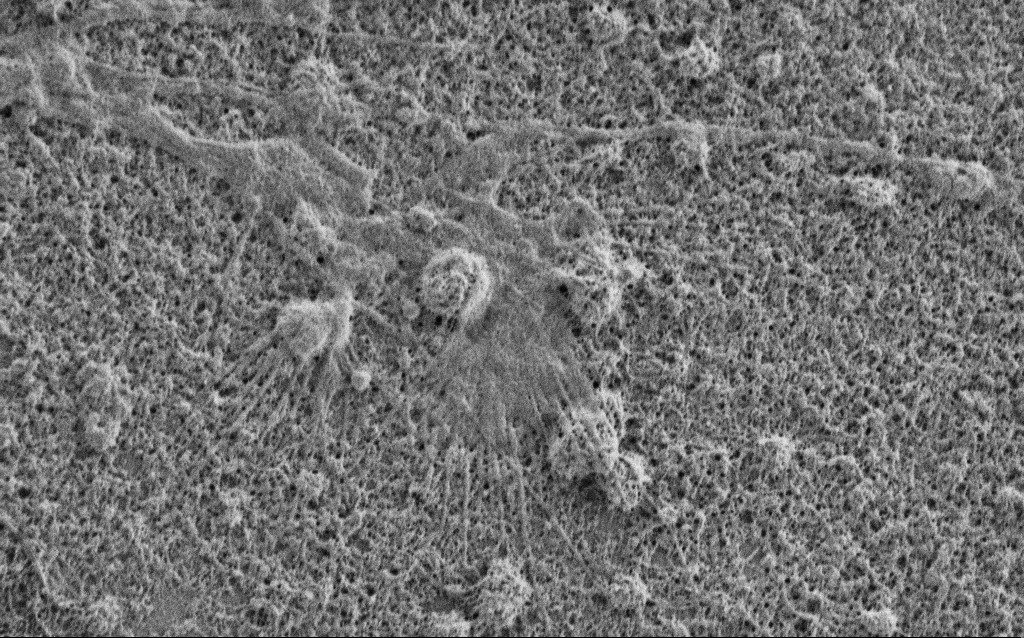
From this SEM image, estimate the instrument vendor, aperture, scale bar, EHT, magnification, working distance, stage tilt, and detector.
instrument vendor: Zeiss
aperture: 30 µm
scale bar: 1000 nm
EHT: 1 kV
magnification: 15 K X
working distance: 5.3 mm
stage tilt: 0°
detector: SE2